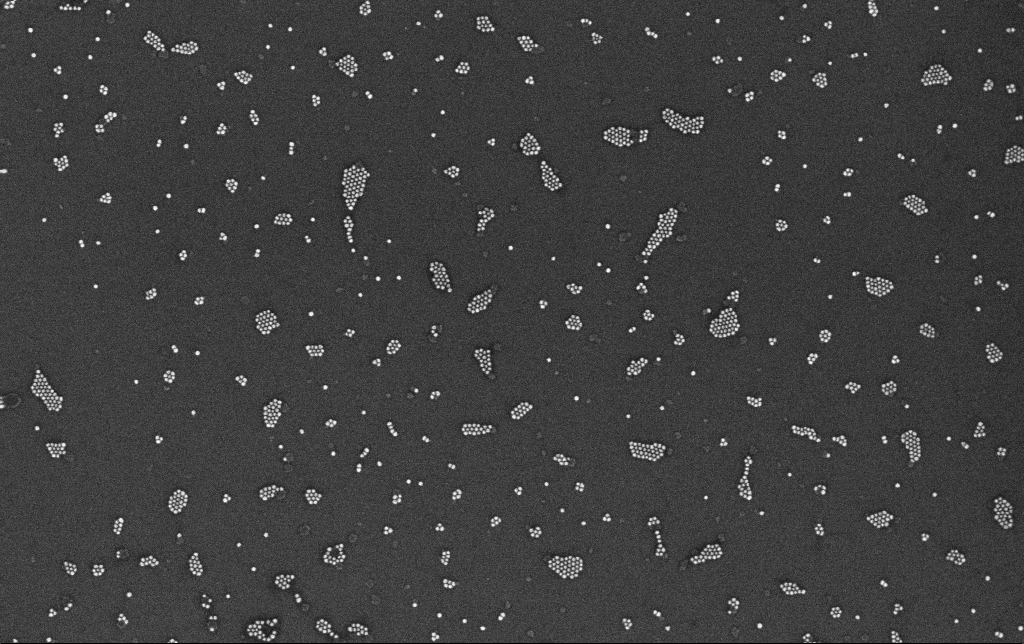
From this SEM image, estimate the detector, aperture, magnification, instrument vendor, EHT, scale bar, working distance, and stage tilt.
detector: InLens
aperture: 30 µm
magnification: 100 K X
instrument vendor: Zeiss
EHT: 10 kV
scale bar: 200 nm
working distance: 3.4 mm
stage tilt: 0°